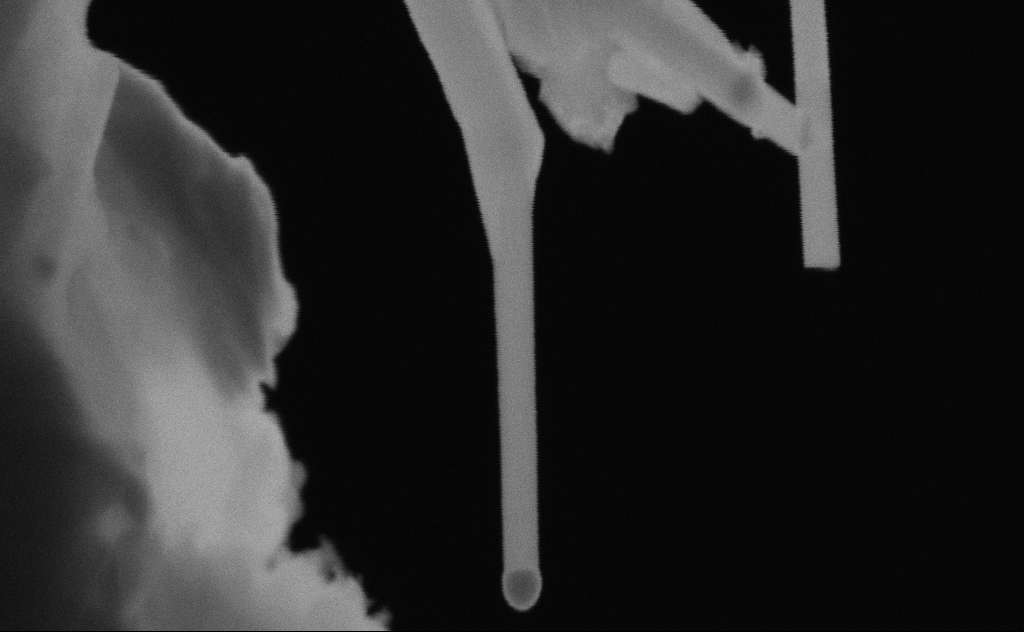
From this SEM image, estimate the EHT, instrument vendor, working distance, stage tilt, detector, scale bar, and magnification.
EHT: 20 kV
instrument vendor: Zeiss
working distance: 9 mm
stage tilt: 0°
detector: SE2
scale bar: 100 nm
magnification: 279.28 K X